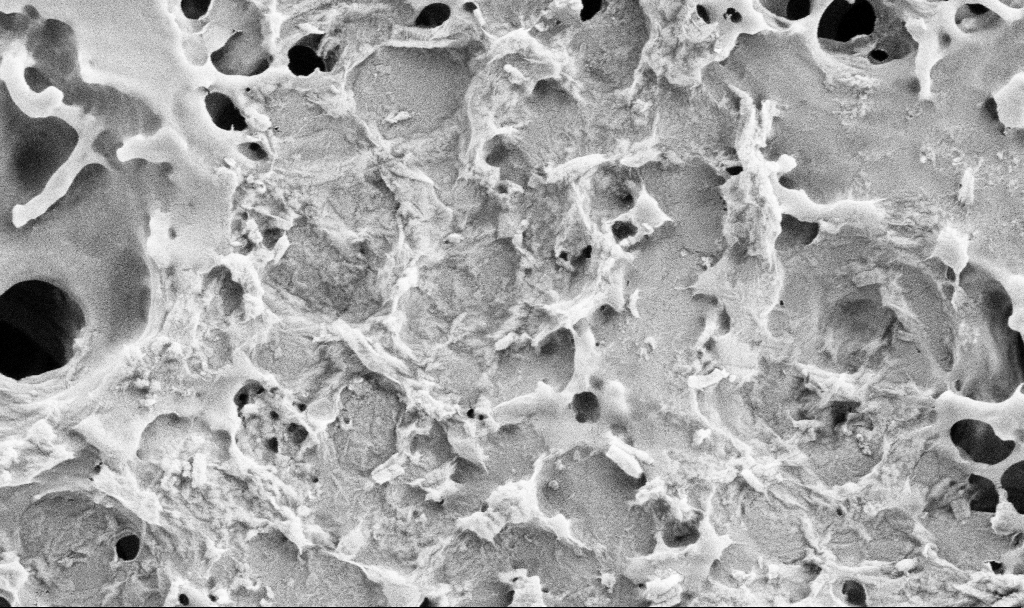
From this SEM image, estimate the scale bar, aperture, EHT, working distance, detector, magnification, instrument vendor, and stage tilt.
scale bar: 1000 nm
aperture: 30 µm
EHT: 2 kV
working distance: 3.6 mm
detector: SE2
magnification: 50 K X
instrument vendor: Zeiss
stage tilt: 0°